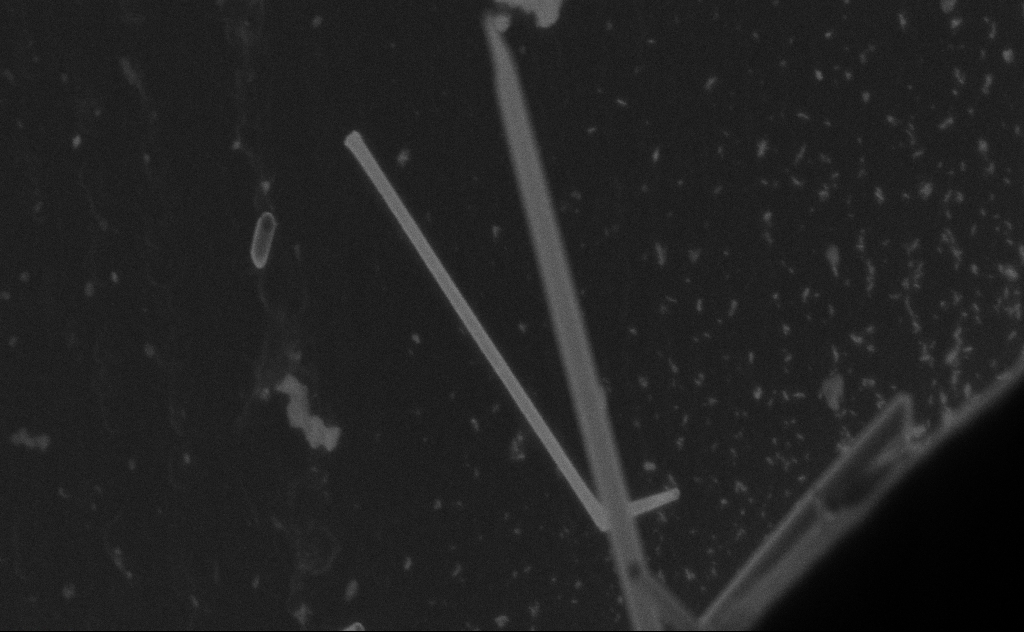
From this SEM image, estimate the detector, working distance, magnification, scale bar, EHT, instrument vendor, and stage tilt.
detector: SE2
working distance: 9 mm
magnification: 99.43 K X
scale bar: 200 nm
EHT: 20 kV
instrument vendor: Zeiss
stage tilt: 0°